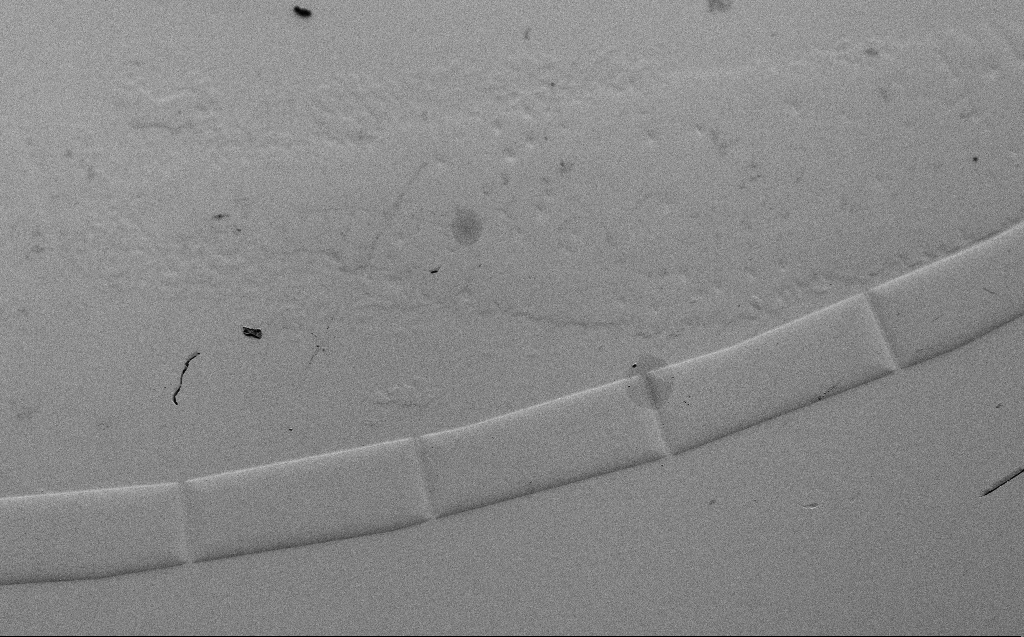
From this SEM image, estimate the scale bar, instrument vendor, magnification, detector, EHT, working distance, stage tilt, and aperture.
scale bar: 200000 nm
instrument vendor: Zeiss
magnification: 0.249 K X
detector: SE2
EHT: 5 kV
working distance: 8 mm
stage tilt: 45°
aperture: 30 µm